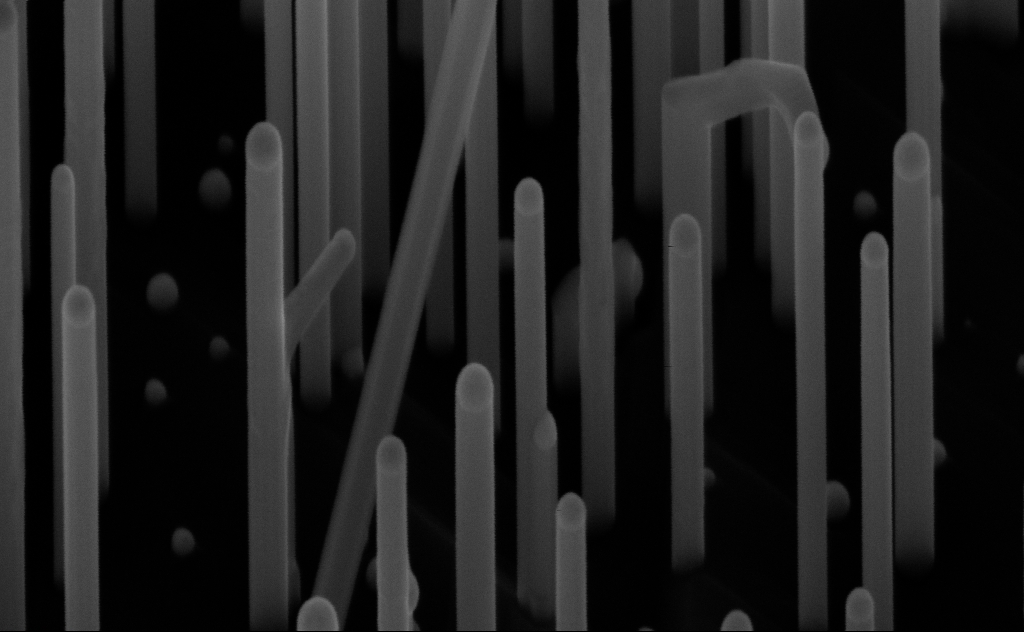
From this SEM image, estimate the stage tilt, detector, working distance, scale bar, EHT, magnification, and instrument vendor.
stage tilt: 45°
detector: InLens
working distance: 7 mm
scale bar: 200 nm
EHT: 10 kV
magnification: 209.66 K X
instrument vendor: Zeiss